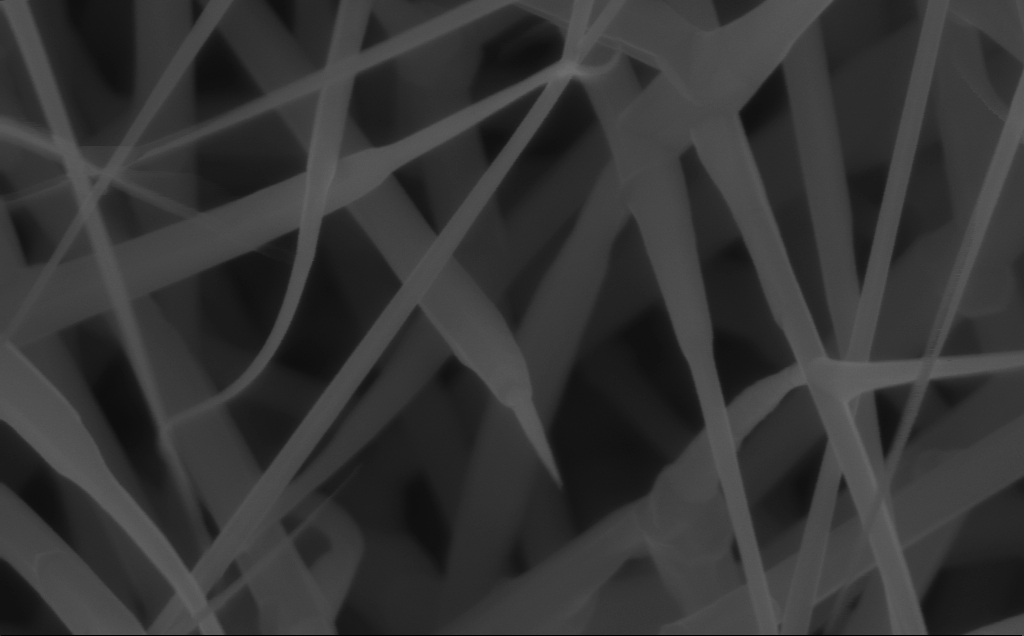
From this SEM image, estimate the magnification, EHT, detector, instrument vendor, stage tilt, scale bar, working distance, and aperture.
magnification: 150 K X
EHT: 10 kV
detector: InLens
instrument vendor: Zeiss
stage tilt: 0°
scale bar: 200 nm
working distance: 6 mm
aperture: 30 µm